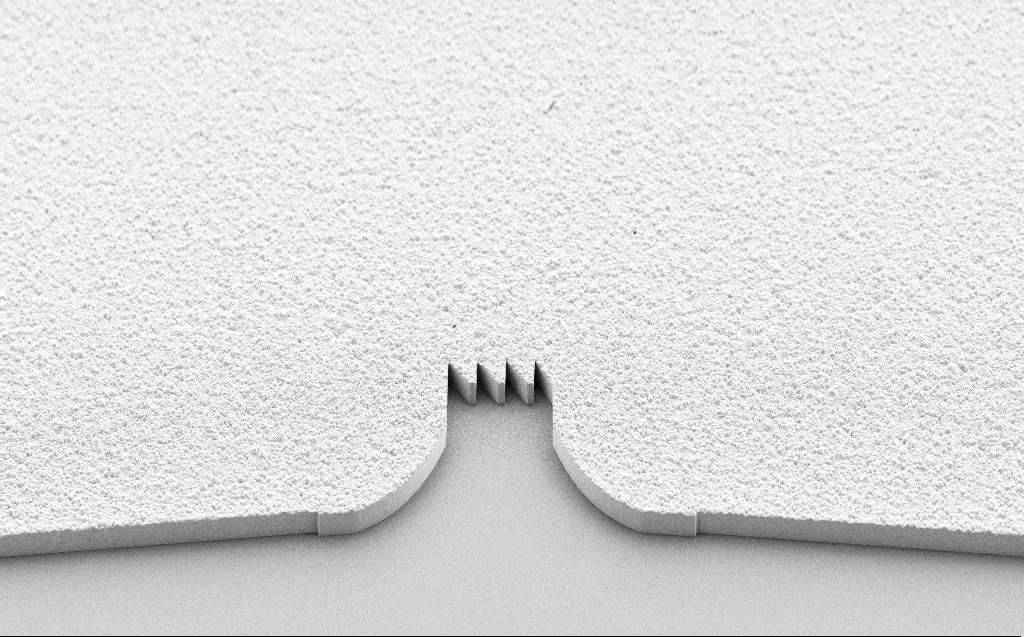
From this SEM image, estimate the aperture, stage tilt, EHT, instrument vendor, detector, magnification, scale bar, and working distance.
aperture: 30 µm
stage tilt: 45°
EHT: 5 kV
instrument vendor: Zeiss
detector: SE2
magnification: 0.934 K X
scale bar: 20000 nm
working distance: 6 mm